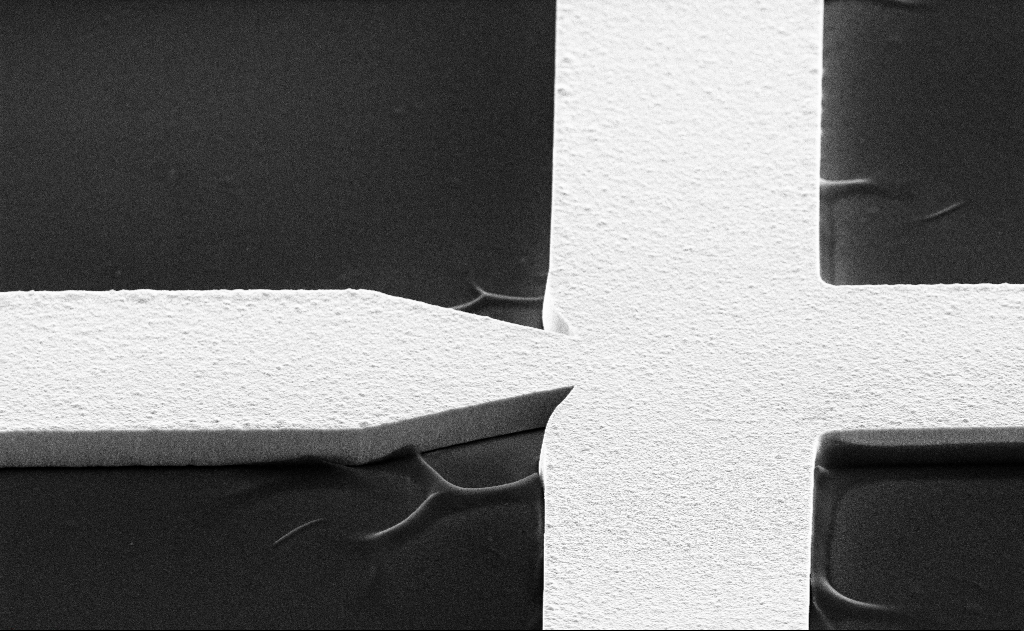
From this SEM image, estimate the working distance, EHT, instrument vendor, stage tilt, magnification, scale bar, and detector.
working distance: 13 mm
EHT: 10 kV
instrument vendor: Zeiss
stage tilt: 60°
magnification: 8.88 K X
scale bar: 2000 nm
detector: SE2